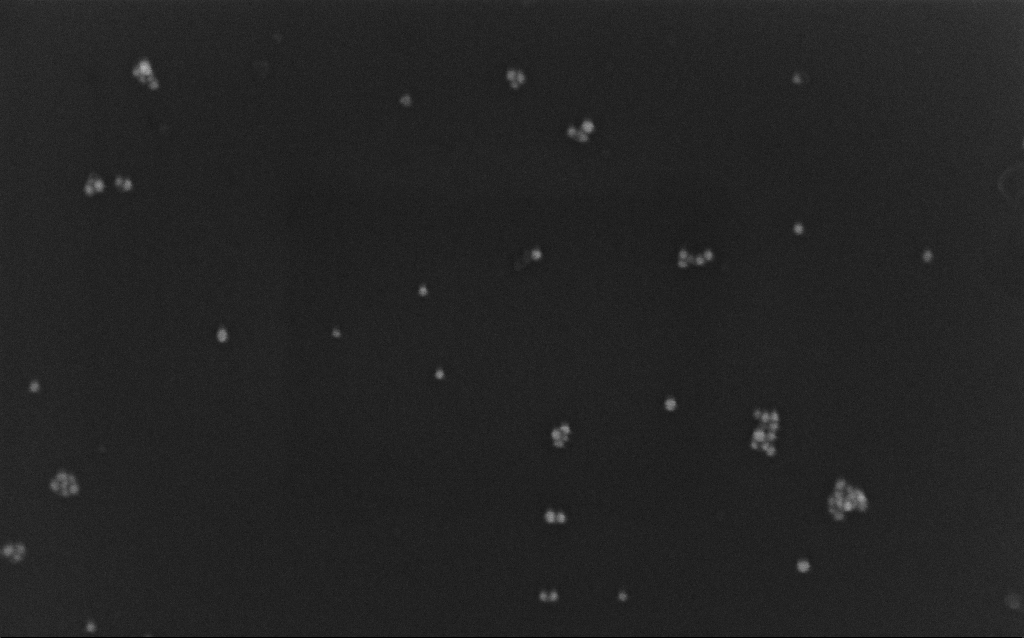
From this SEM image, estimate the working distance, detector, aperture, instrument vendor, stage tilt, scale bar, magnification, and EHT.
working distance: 7 mm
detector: InLens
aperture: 30 µm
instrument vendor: Zeiss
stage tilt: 0°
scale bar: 100 nm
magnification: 200.27 K X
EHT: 10 kV